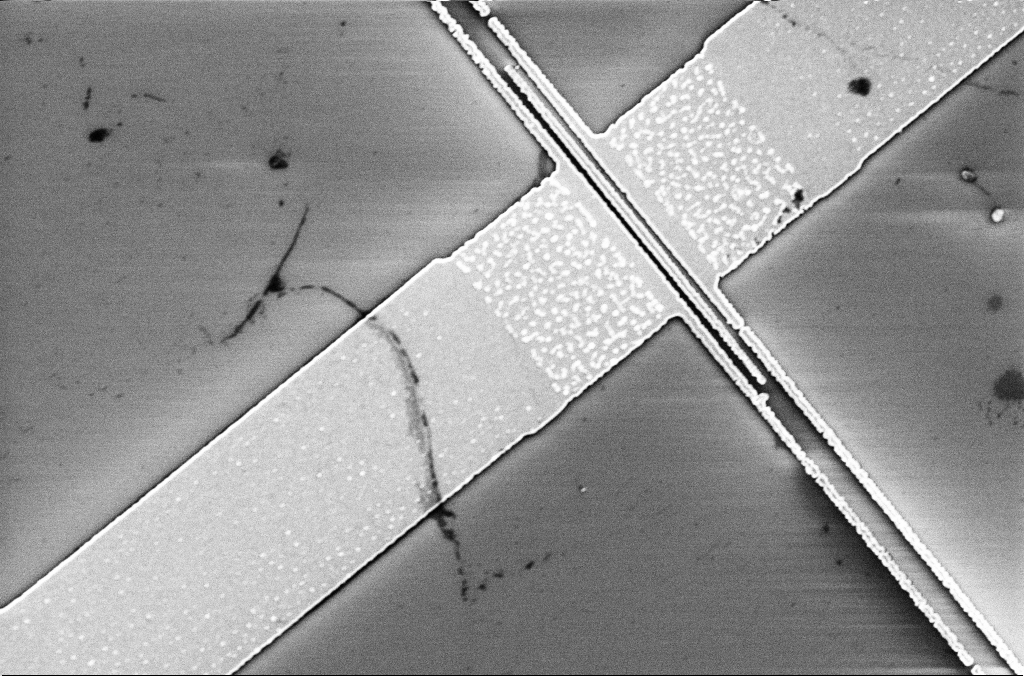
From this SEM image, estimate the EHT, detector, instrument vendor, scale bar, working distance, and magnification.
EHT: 5 kV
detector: InLens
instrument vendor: Zeiss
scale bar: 2000 nm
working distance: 3.3 mm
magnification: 14.16 K X